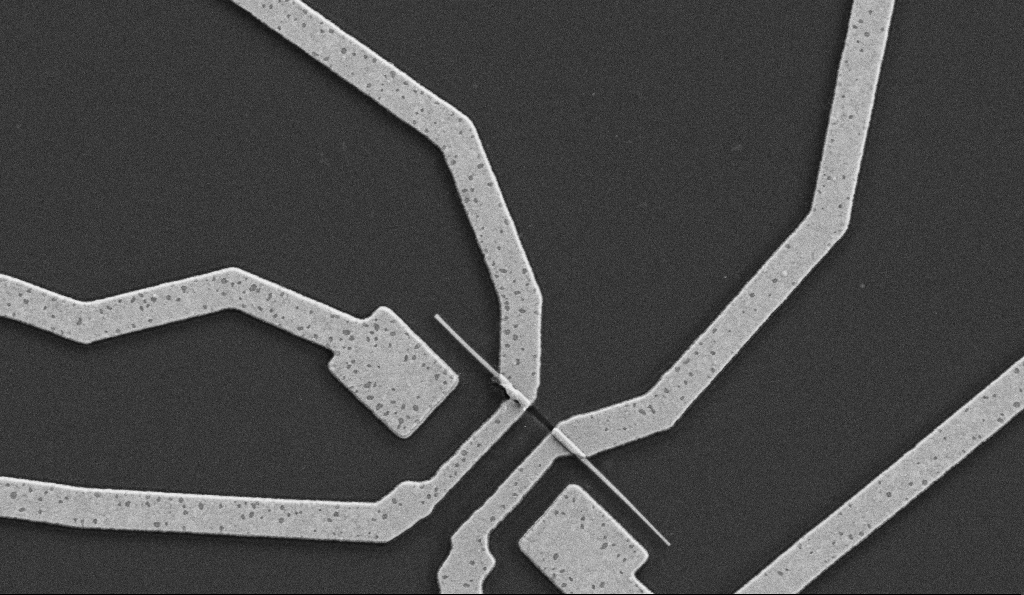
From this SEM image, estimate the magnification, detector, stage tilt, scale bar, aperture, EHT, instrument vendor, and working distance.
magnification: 20 K X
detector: SE2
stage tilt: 0°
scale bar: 1000 nm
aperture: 30 µm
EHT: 5 kV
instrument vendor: Zeiss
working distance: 7.7 mm